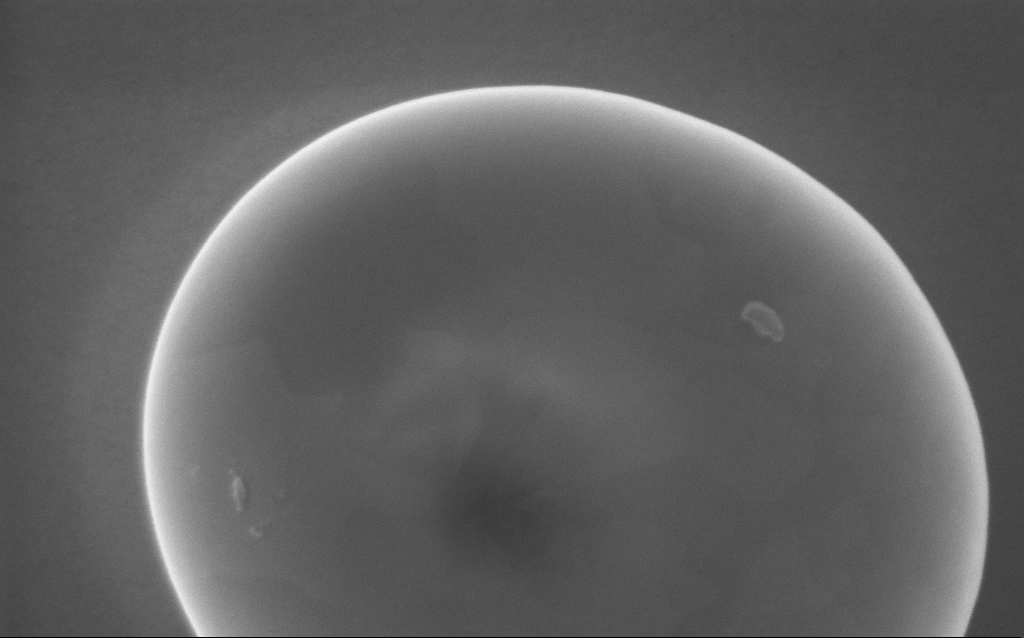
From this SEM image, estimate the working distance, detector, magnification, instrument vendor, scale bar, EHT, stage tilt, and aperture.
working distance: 3 mm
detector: InLens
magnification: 180 K X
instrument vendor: Zeiss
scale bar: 200 nm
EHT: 5 kV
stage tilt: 0°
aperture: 30 µm